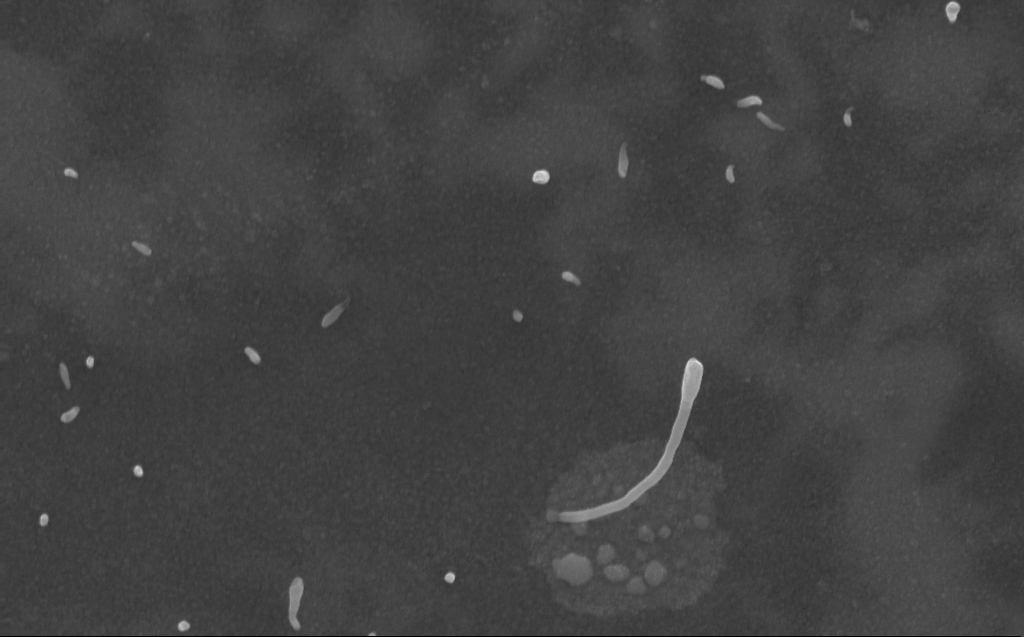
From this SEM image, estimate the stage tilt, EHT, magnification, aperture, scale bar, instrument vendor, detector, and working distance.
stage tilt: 0°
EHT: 10 kV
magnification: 58.89 K X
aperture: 30 µm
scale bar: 1000 nm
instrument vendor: Zeiss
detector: InLens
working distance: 3 mm